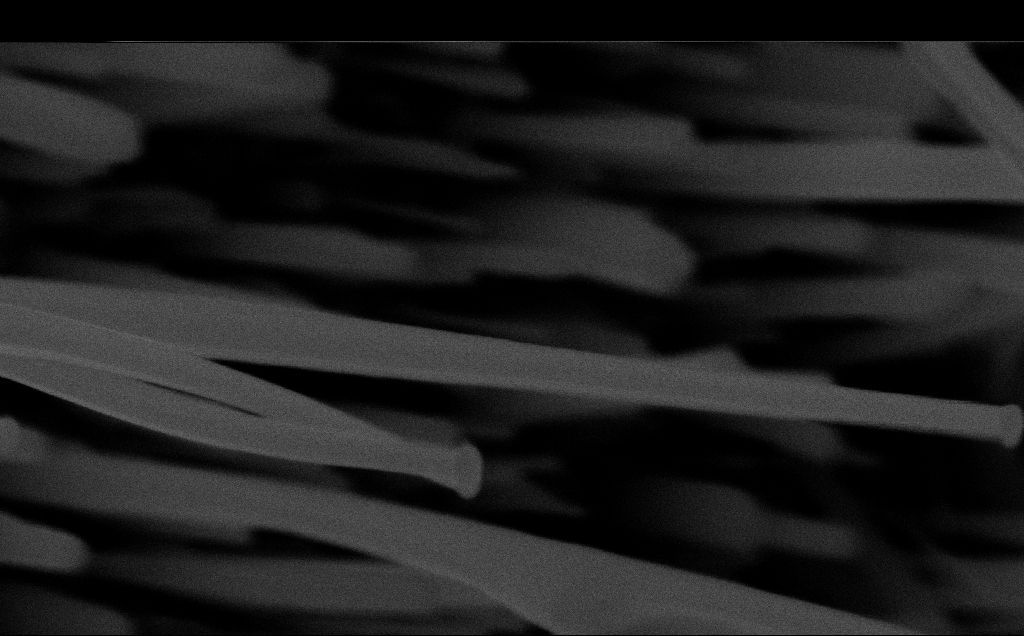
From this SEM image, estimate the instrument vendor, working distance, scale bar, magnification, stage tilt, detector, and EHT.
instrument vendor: Zeiss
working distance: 6 mm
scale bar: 200 nm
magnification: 110.93 K X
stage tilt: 0°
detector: InLens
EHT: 10 kV